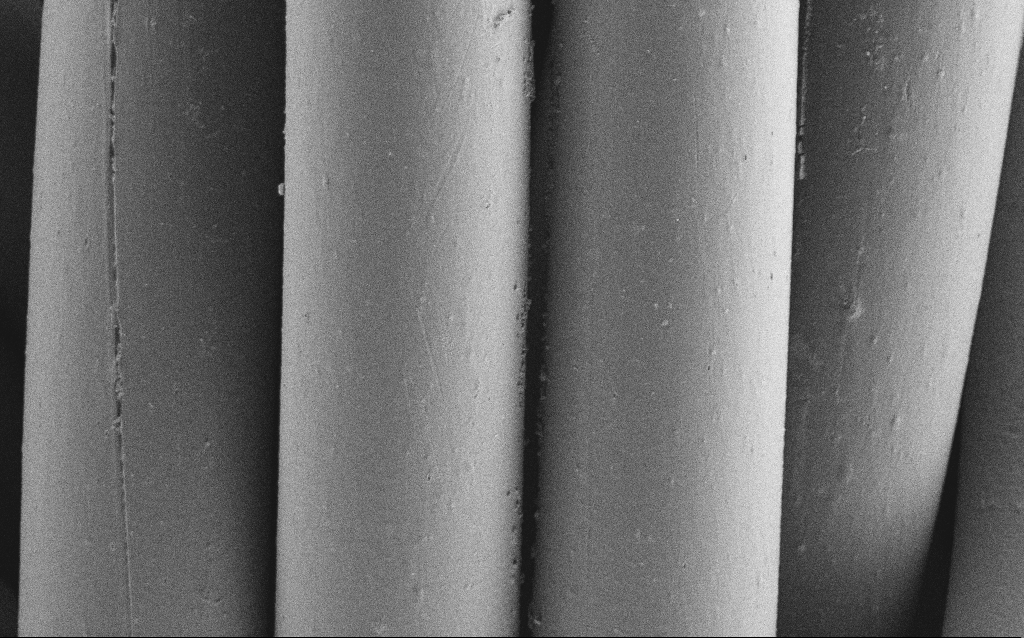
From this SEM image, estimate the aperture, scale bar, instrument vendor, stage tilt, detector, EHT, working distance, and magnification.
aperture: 30 µm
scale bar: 10000 nm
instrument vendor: Zeiss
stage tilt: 0°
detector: SE2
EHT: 1 kV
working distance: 4 mm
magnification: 3.83 K X